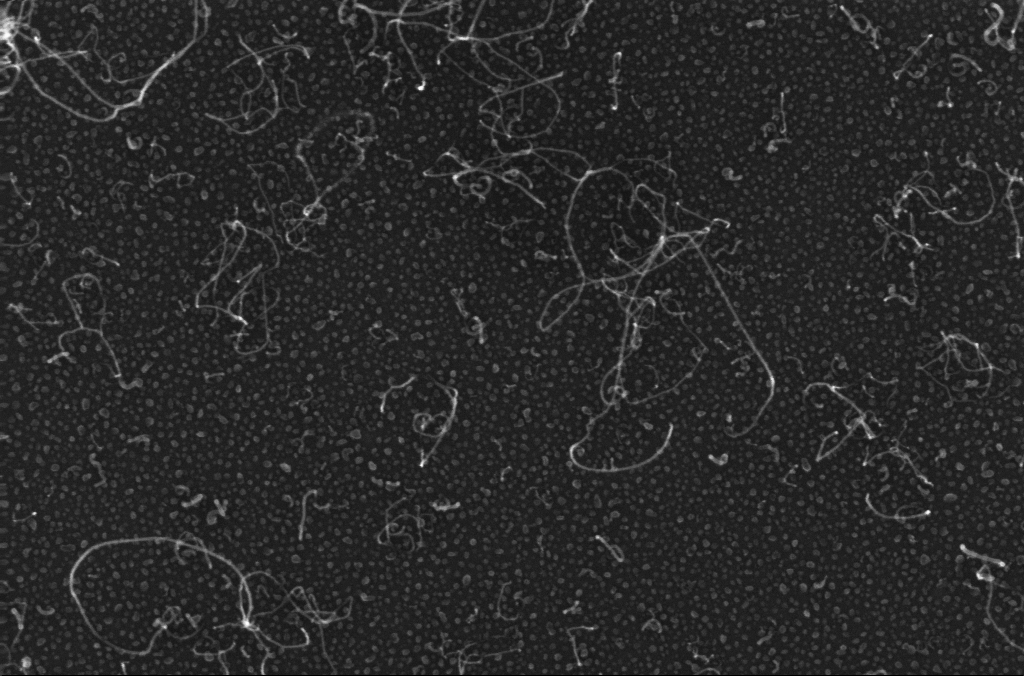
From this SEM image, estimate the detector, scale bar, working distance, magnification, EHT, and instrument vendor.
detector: InLens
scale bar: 100 nm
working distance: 3.2 mm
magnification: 150 K X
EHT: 10 kV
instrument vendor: Zeiss